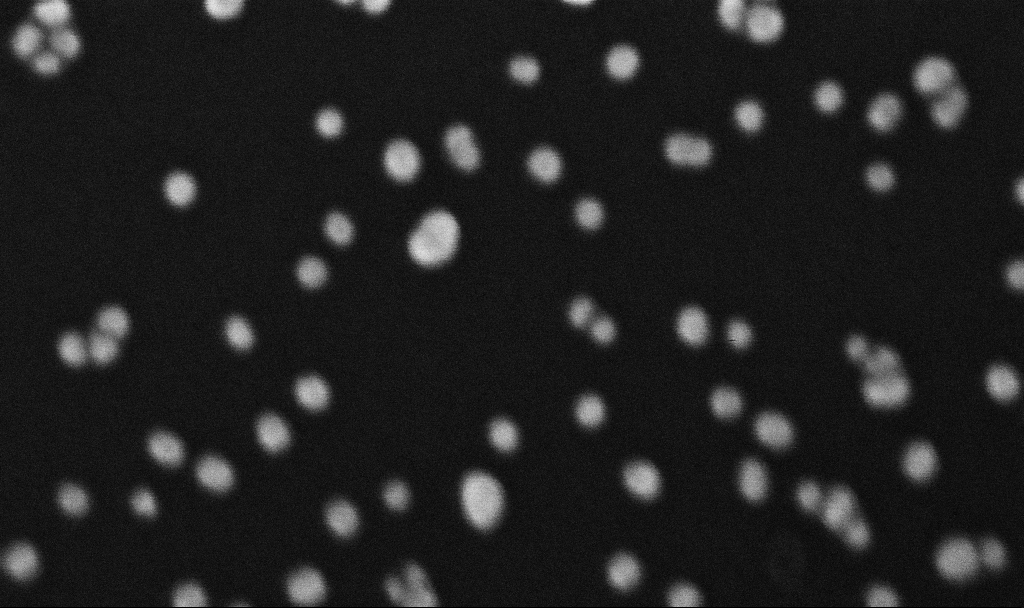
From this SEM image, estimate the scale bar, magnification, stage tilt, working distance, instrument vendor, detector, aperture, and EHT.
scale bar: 100 nm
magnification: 573.58 K X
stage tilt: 0°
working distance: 5.4 mm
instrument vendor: Zeiss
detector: InLens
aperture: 30 µm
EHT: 10 kV